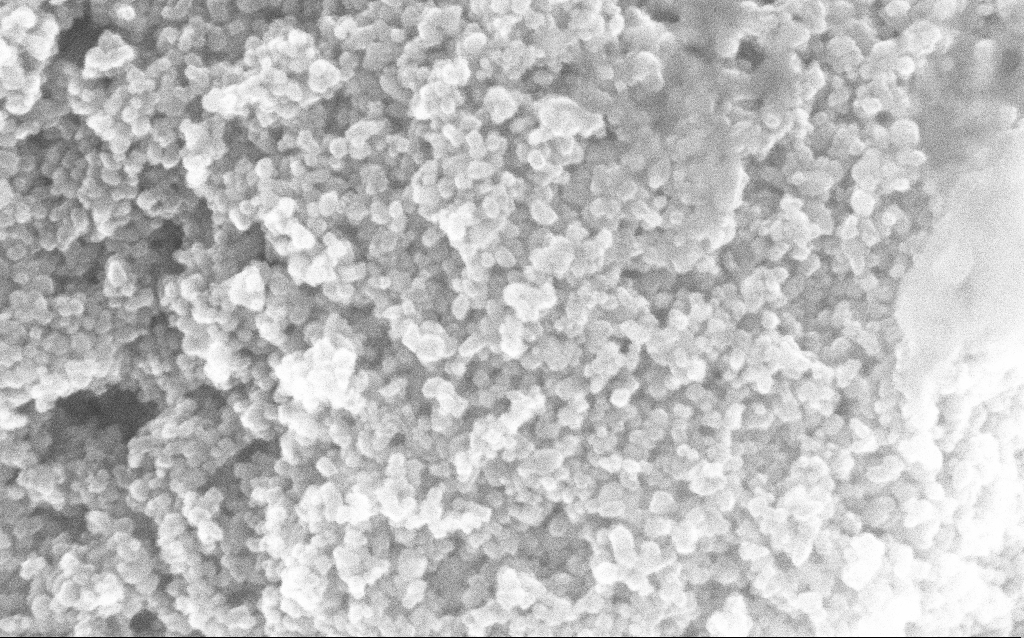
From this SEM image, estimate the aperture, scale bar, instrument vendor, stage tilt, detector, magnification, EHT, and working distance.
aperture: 30 µm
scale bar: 100 nm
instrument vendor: Zeiss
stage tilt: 0°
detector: InLens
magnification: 239.68 K X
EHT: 10 kV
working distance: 3.8 mm